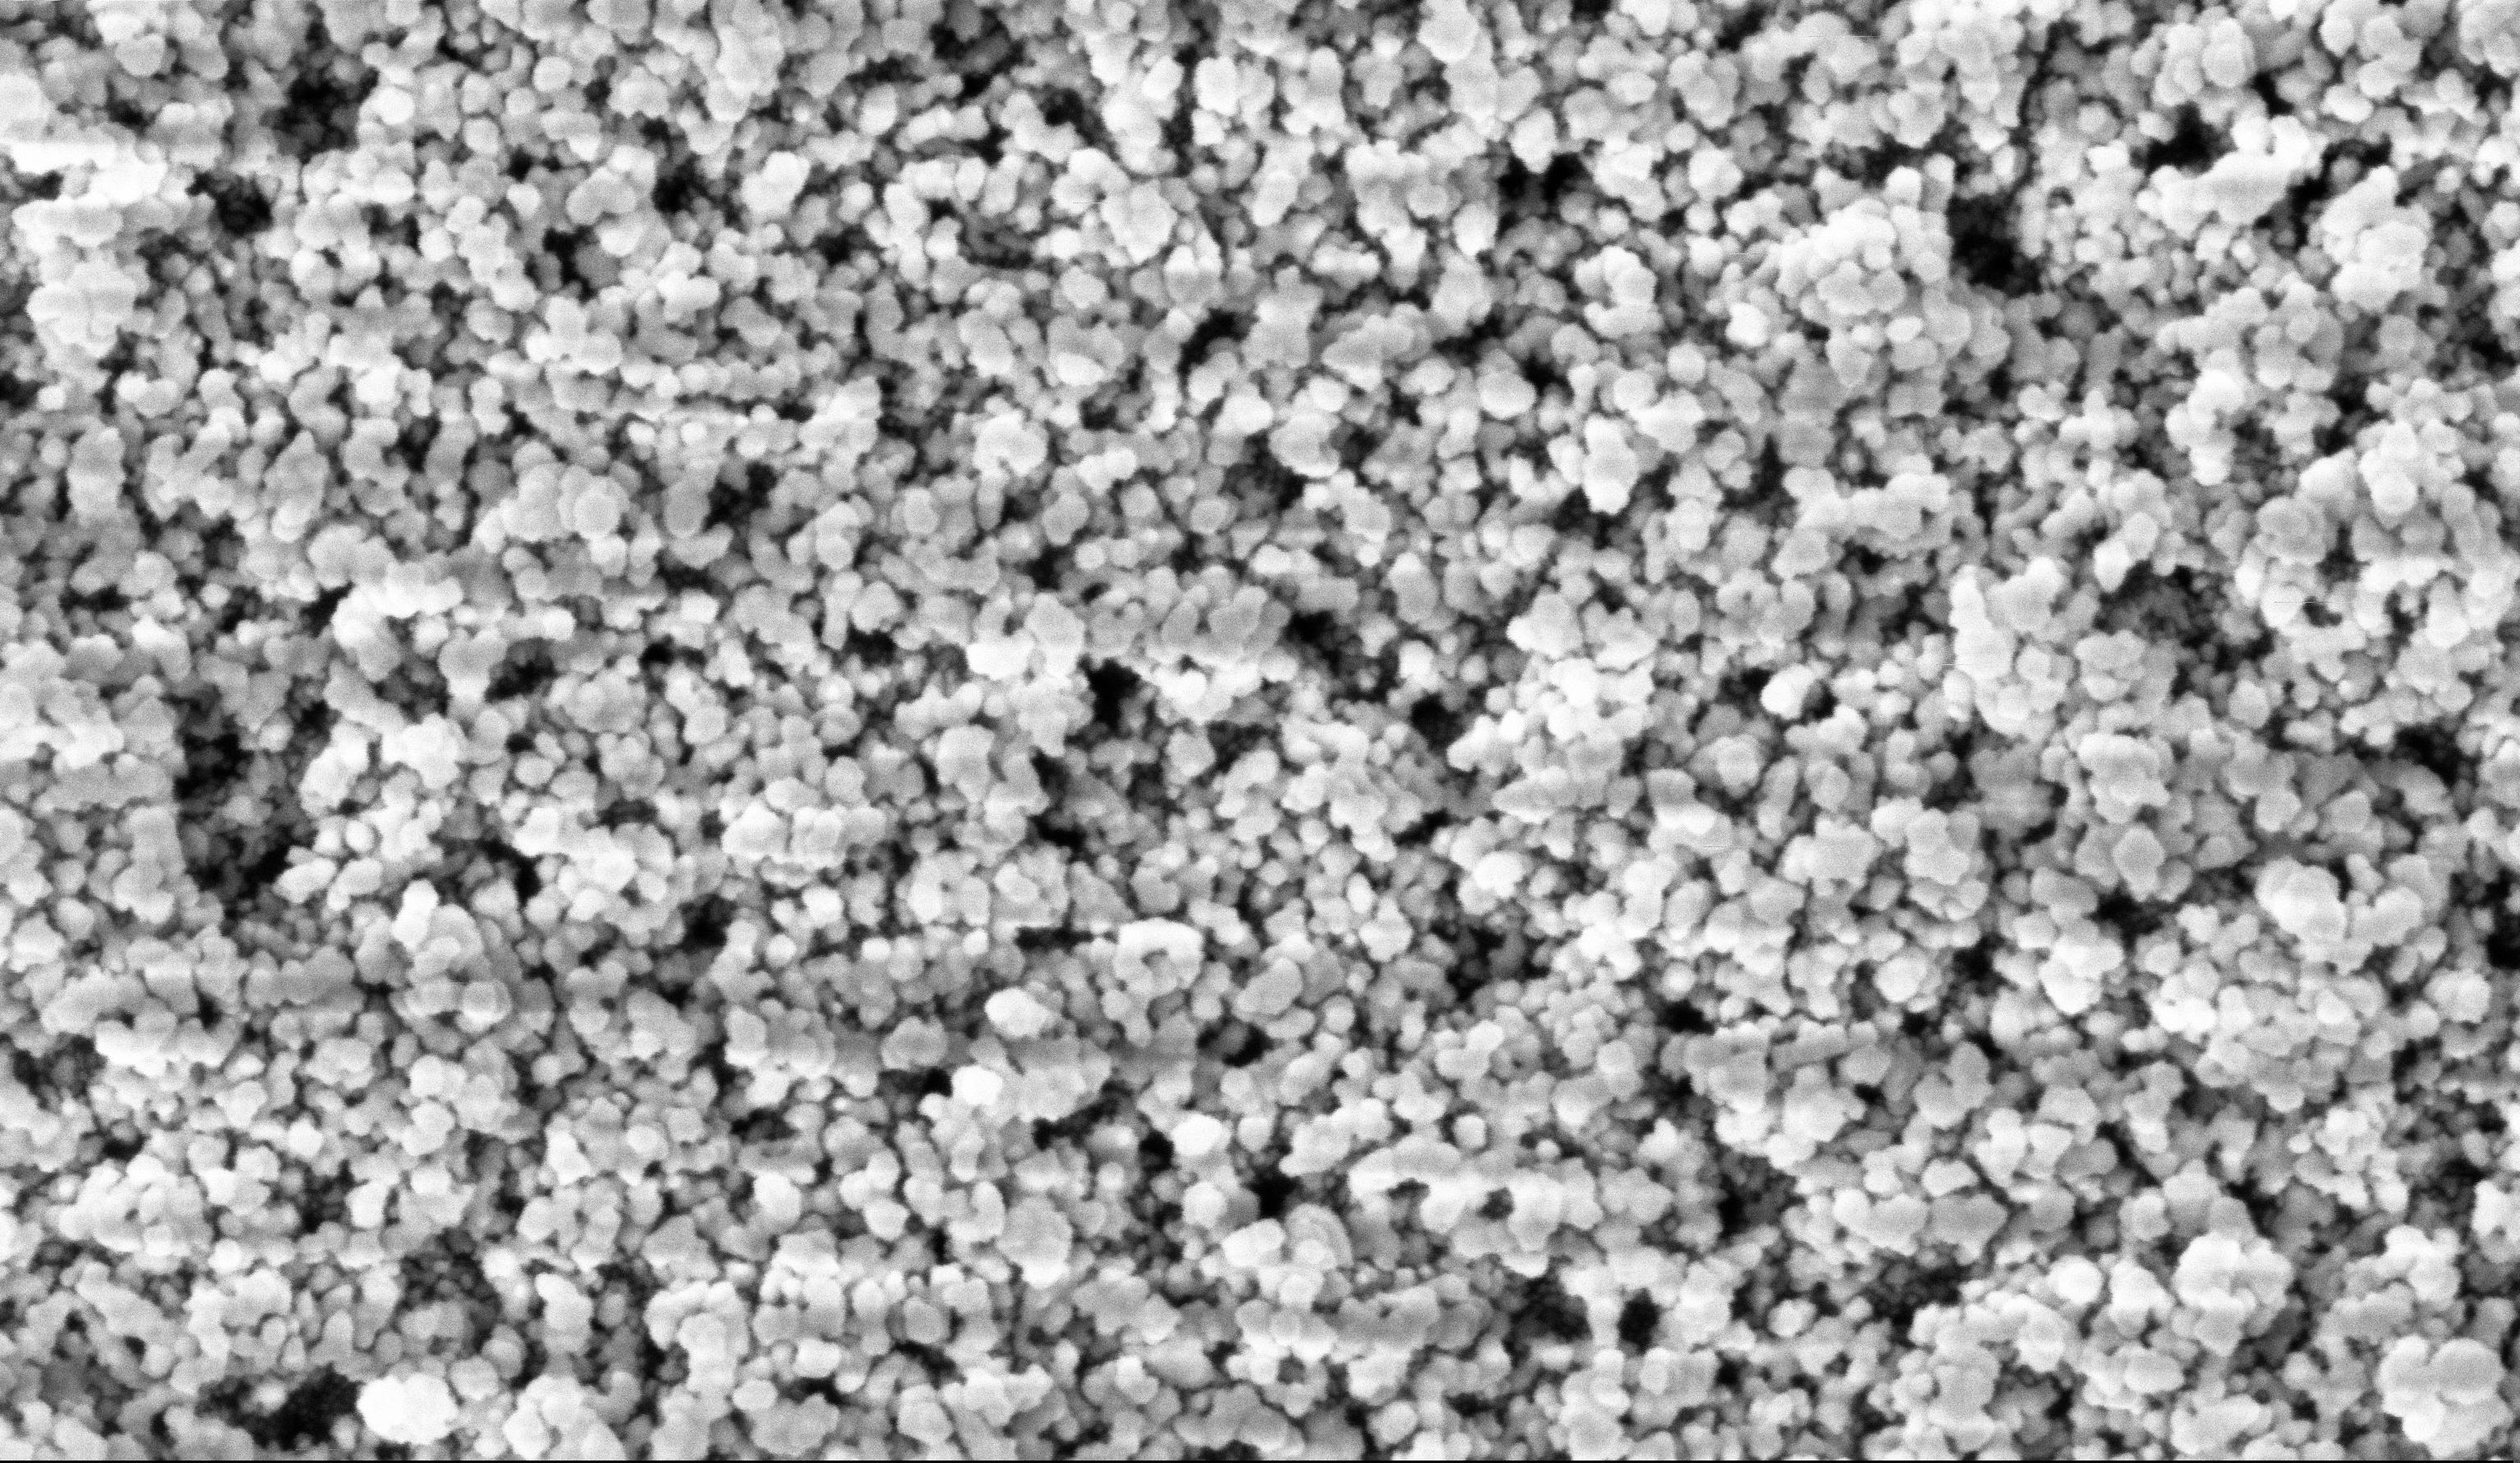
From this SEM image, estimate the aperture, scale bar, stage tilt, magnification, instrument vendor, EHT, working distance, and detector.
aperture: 30 µm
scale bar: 100 nm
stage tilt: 0°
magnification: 135 K X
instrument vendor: Zeiss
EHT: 5 kV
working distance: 6 mm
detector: InLens